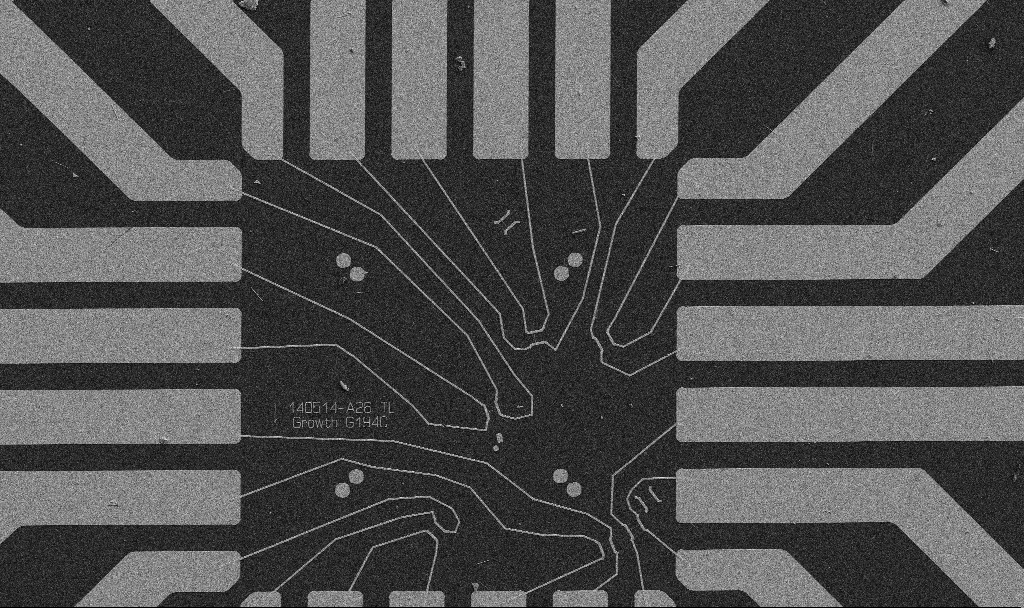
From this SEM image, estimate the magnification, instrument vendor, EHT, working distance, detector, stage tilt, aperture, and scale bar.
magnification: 1 K X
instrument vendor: Zeiss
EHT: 5 kV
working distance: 10.7 mm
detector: SE2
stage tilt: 0°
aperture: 30 µm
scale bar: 20000 nm